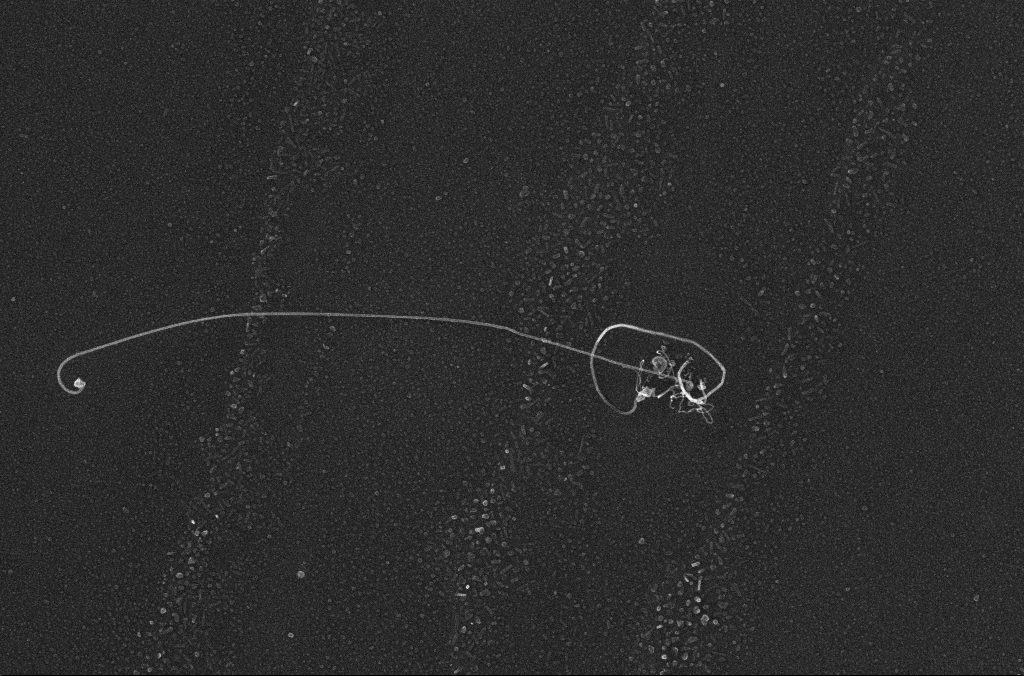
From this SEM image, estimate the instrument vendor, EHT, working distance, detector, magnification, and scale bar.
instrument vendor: Zeiss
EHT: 10 kV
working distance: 3.3 mm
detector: InLens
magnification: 50 K X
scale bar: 1000 nm